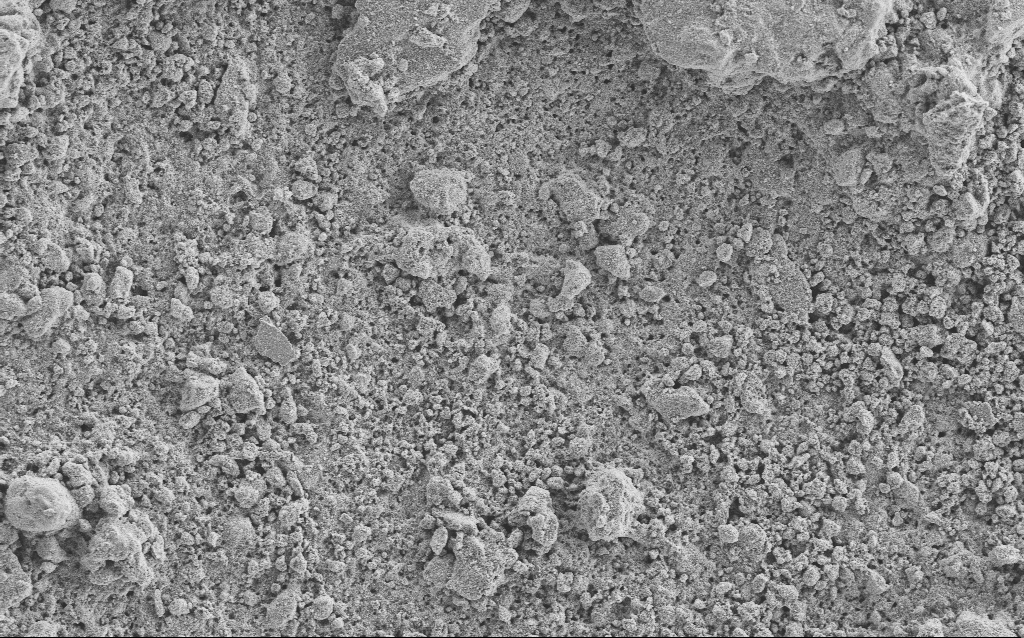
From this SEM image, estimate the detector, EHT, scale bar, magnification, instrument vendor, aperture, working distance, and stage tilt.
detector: SE2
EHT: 5 kV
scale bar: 10000 nm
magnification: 1.23 K X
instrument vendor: Zeiss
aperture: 30 µm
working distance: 4.7 mm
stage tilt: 0°